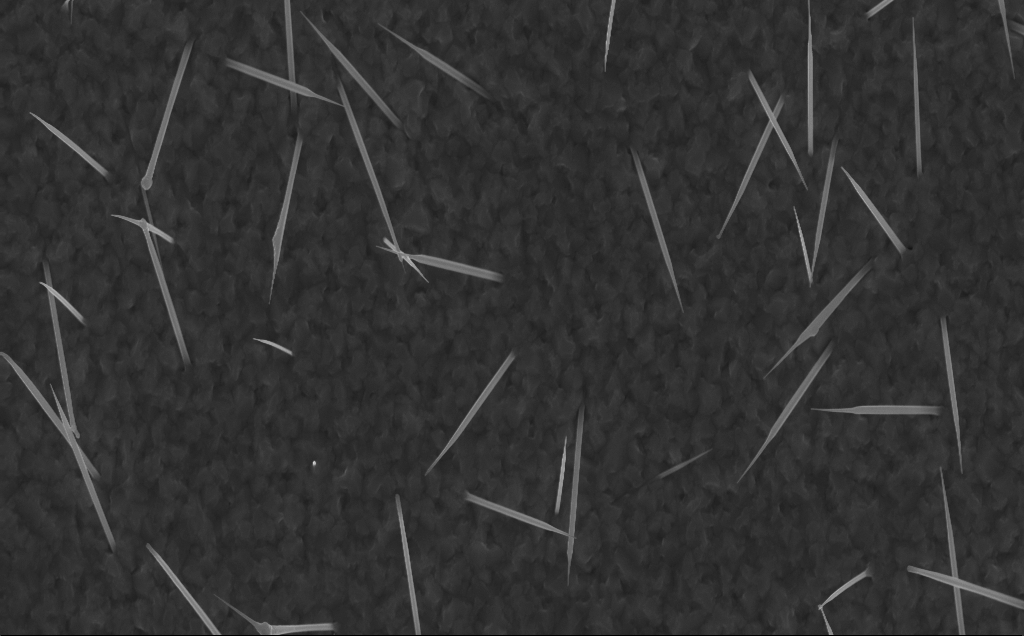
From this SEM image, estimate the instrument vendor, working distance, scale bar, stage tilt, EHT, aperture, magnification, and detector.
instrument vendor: Zeiss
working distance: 5 mm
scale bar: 2000 nm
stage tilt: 0°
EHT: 10 kV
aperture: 30 µm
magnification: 20 K X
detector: InLens